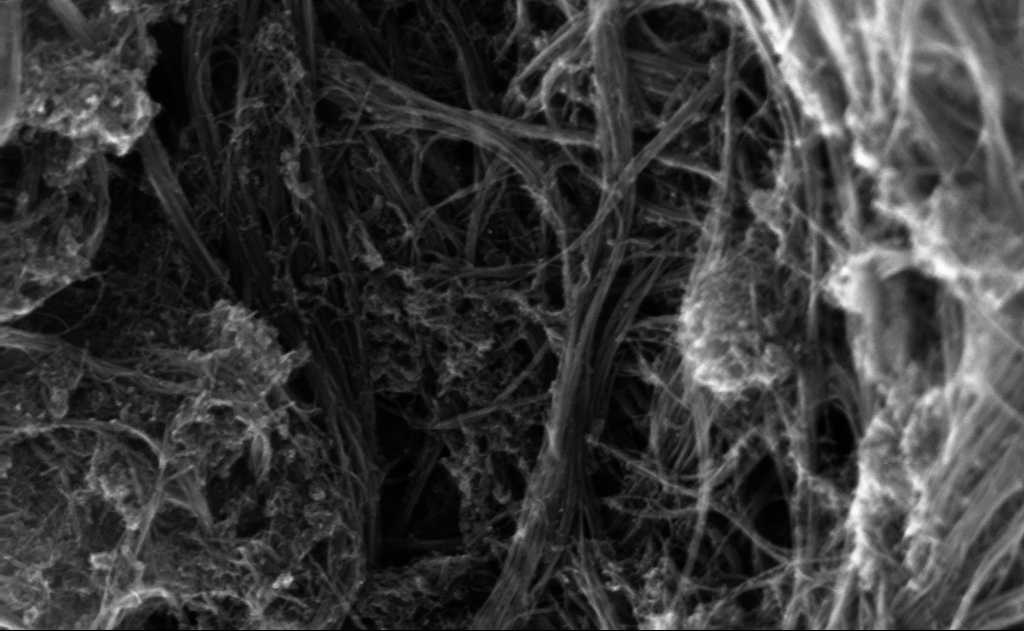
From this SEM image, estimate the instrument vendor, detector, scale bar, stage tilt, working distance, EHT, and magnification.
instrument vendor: Zeiss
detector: InLens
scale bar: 100 nm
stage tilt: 0°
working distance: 3 mm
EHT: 10 kV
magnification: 237.59 K X